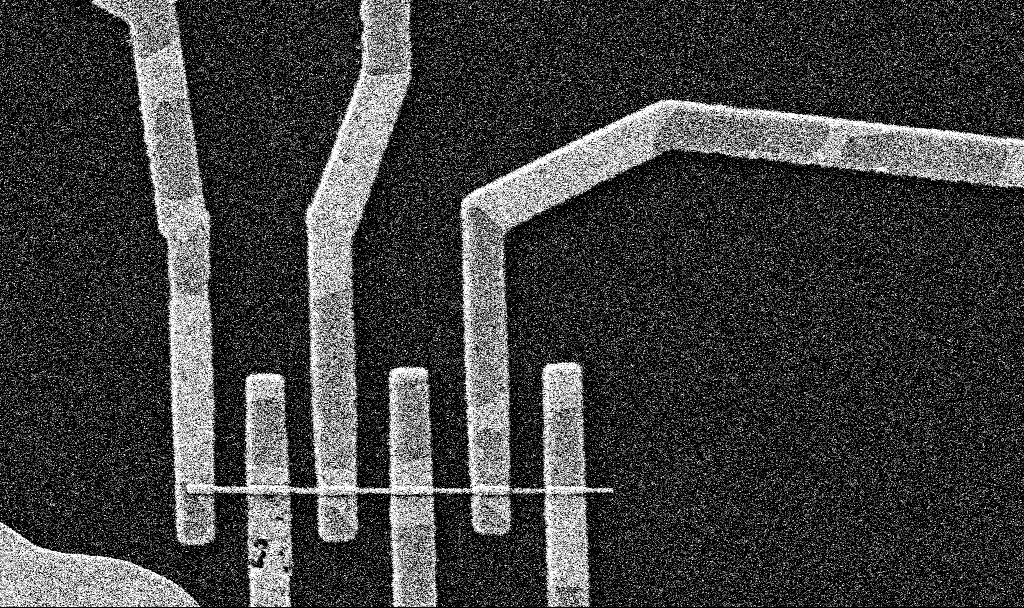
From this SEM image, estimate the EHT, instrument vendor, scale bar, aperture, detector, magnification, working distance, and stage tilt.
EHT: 5 kV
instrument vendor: Zeiss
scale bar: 2000 nm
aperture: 30 µm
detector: SE2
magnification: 24.13 K X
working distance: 8.5 mm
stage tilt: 0°